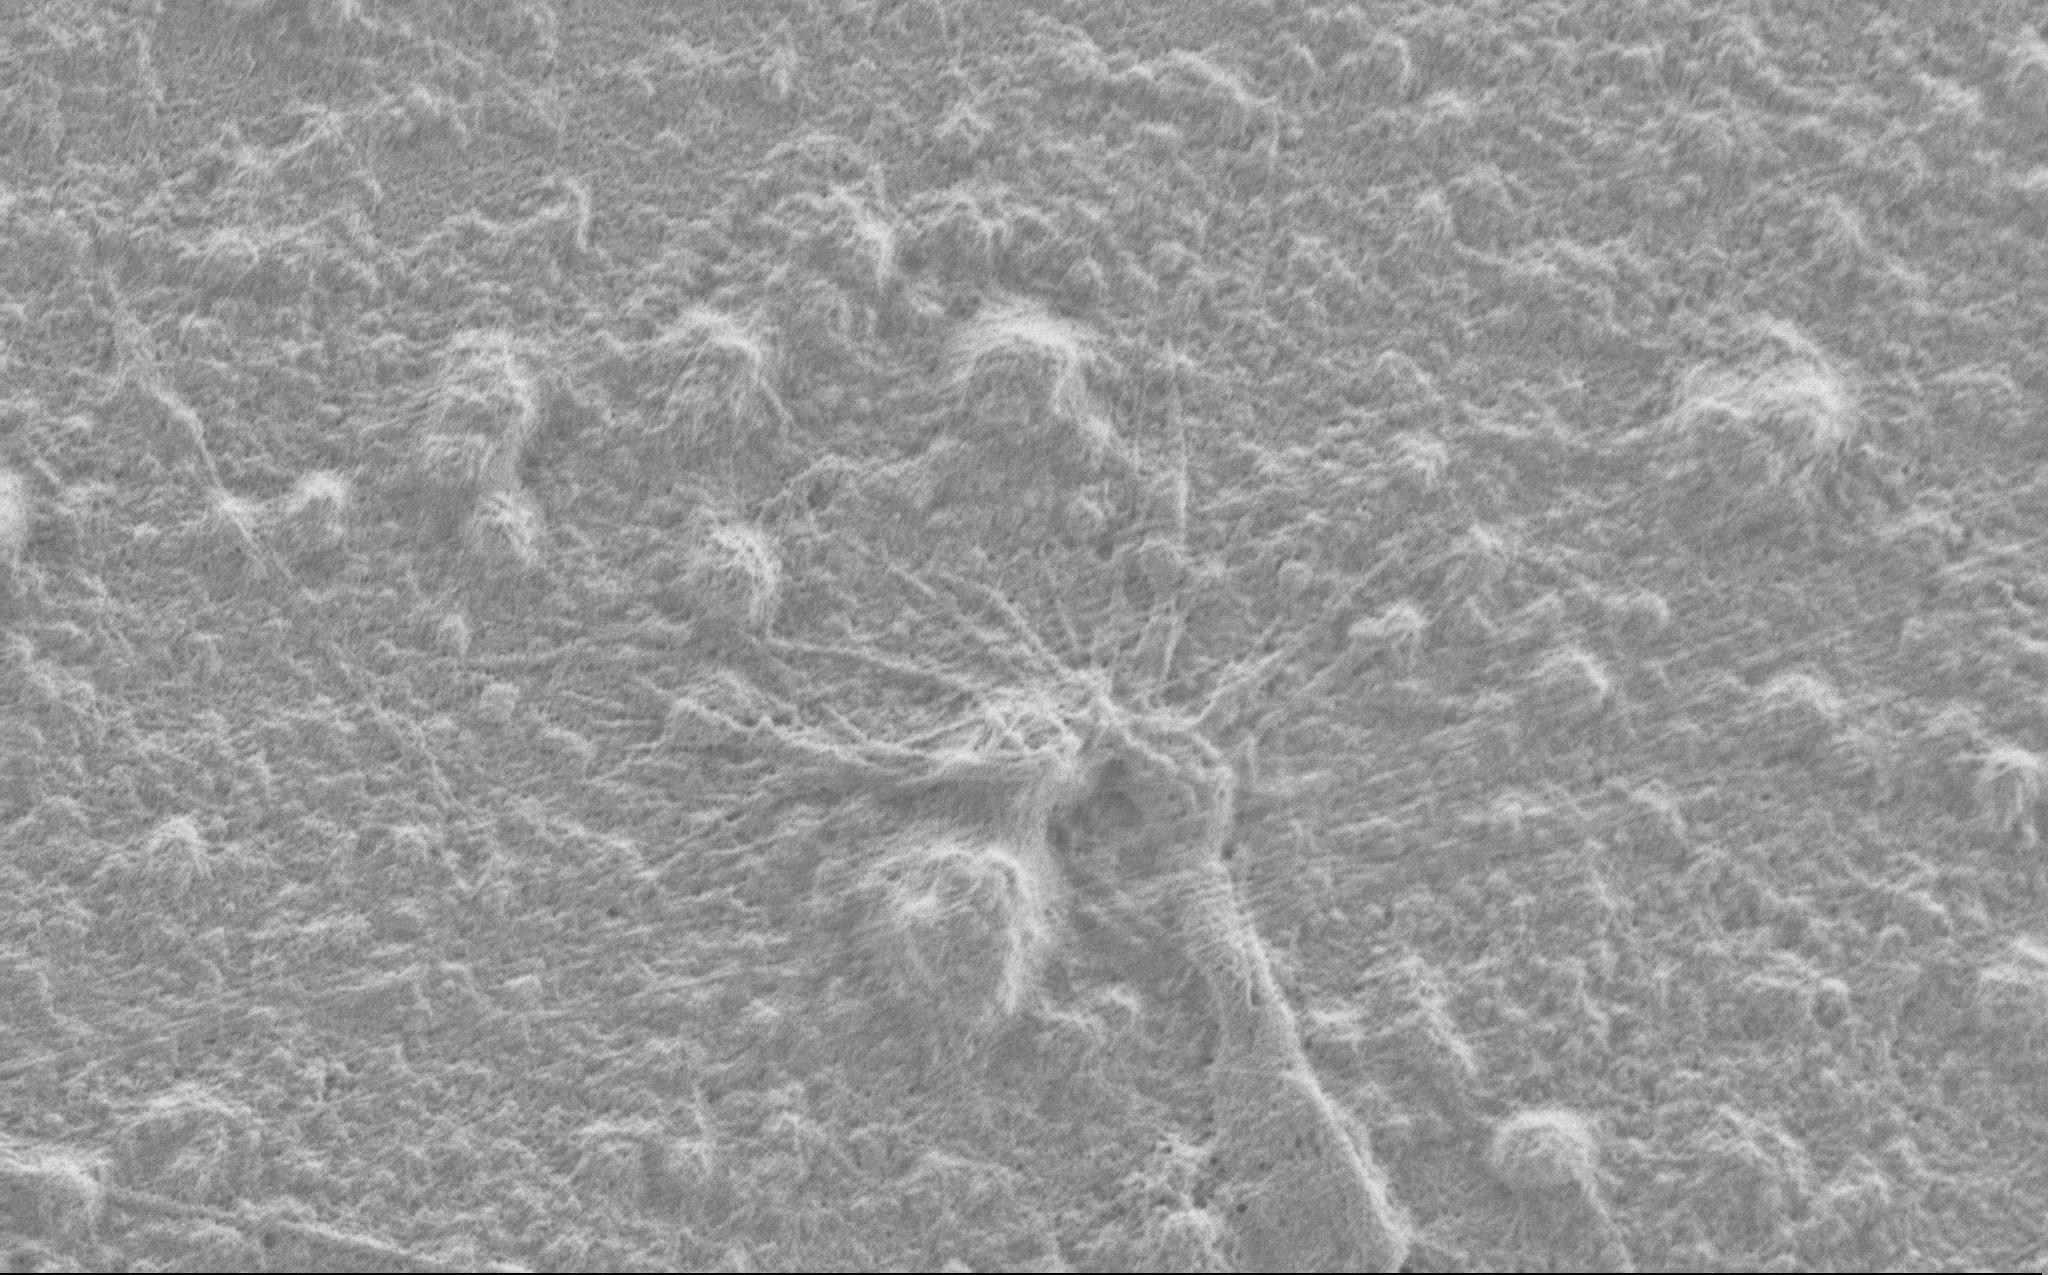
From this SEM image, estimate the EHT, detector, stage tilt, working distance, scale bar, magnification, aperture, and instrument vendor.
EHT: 0.9 kV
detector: SE2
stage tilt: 0°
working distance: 5 mm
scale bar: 2000 nm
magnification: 10 K X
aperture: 30 µm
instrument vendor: Zeiss